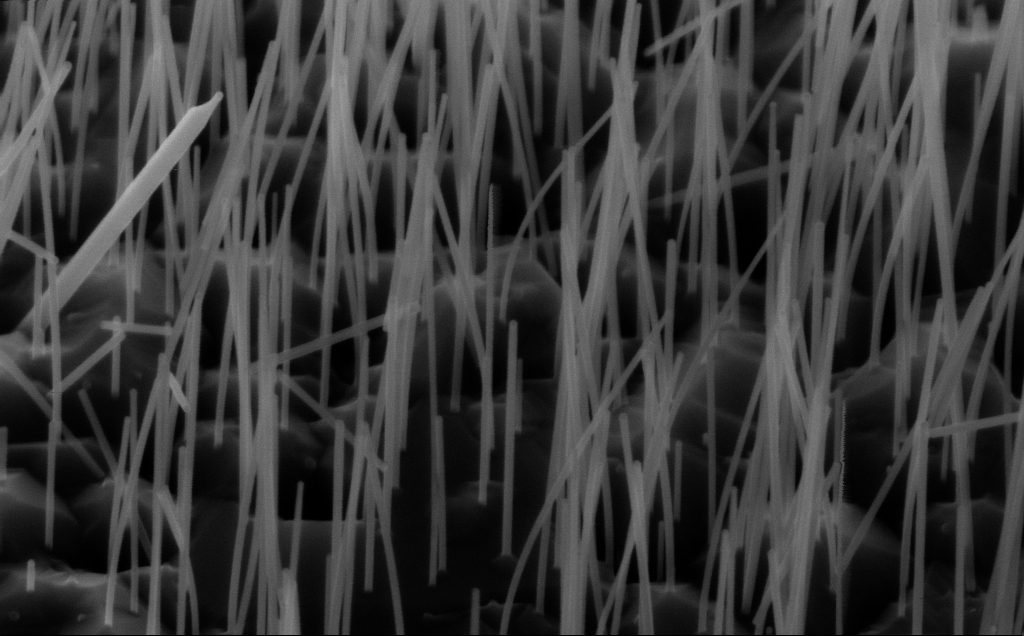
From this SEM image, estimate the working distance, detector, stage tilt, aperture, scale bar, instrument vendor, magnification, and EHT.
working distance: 5 mm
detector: InLens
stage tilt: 45°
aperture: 30 µm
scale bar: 200 nm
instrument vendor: Zeiss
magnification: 80 K X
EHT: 10 kV